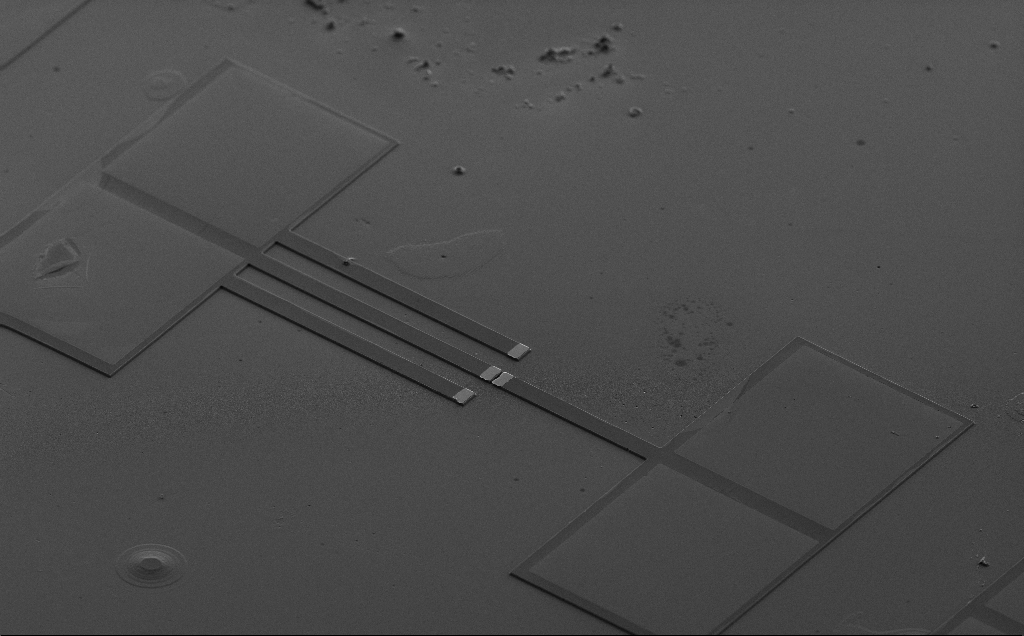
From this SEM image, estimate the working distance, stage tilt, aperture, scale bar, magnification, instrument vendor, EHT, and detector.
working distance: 10 mm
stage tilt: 50°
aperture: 30 µm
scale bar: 100000 nm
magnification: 0.412 K X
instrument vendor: Zeiss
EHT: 5 kV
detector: SE2